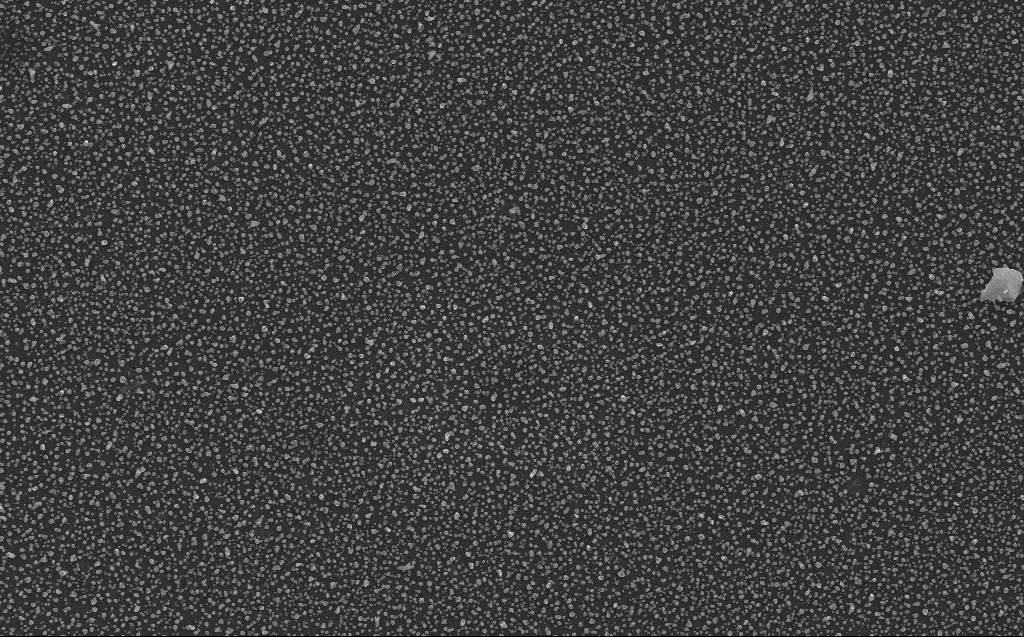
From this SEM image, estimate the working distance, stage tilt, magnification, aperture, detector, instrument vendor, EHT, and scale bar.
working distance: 3 mm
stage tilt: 0°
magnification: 10 K X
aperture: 30 µm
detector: InLens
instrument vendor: Zeiss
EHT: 10 kV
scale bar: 2000 nm